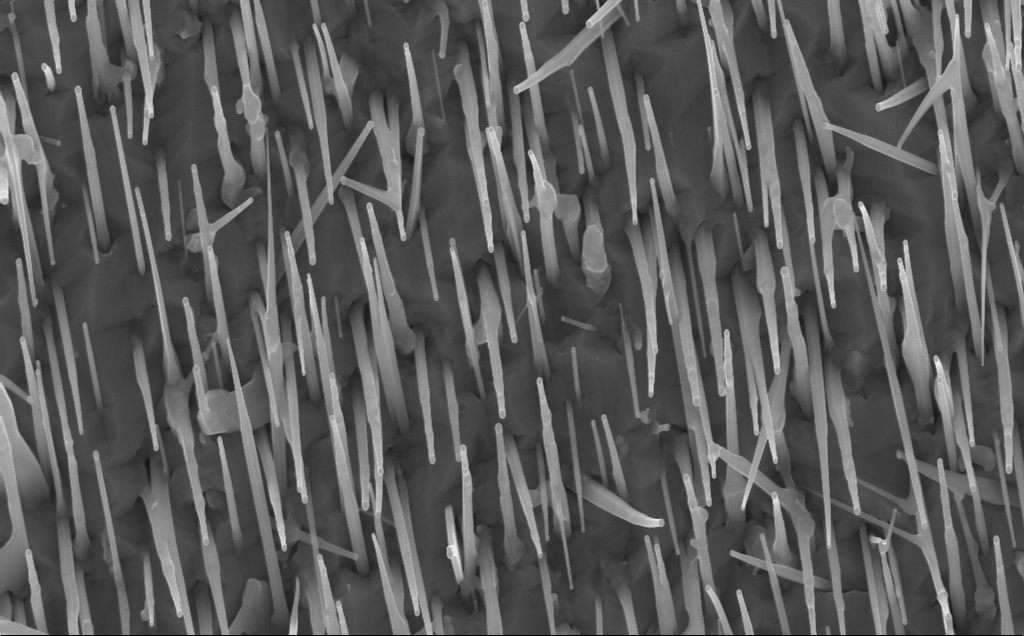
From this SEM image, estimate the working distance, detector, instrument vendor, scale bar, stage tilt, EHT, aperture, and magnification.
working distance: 7 mm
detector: InLens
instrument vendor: Zeiss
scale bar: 1000 nm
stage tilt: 0°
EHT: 10 kV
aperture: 30 µm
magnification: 40 K X